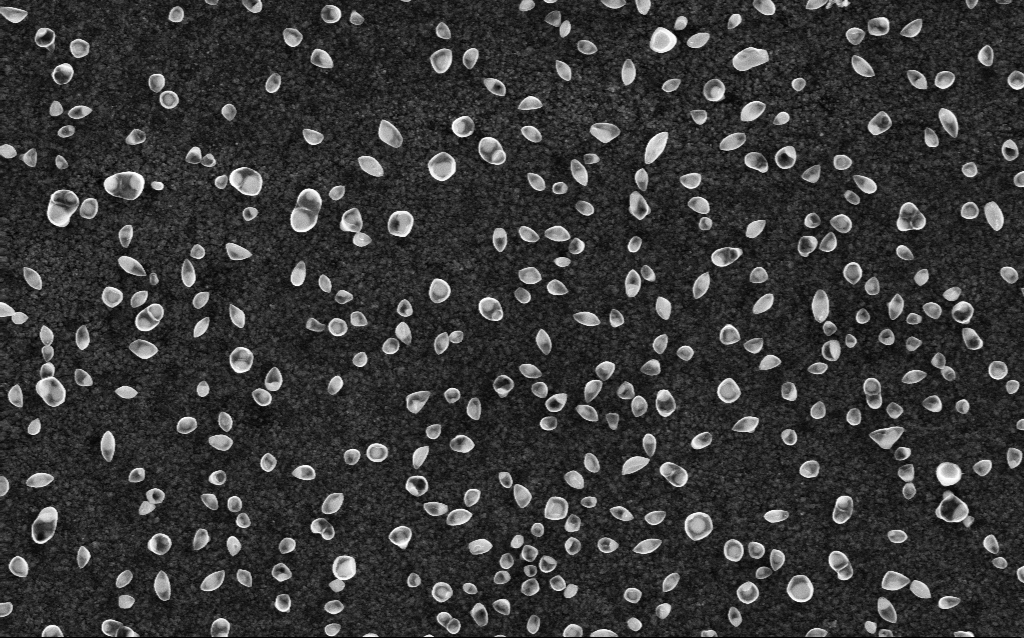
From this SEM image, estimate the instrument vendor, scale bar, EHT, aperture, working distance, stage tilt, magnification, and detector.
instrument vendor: Zeiss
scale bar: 1000 nm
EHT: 5 kV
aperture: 30 µm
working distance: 2.6 mm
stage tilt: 0°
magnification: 50 K X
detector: InLens